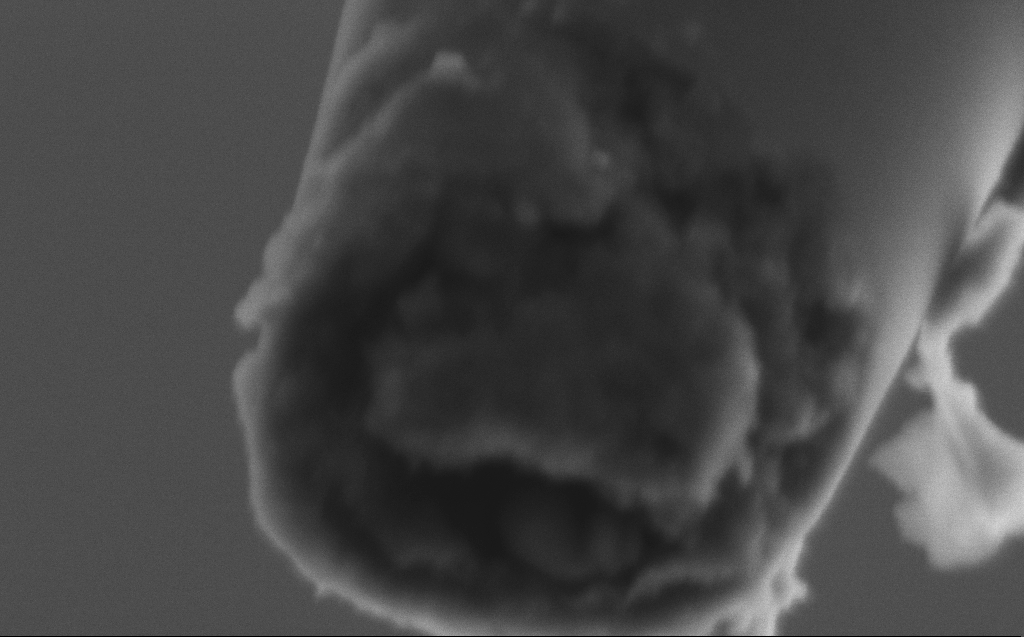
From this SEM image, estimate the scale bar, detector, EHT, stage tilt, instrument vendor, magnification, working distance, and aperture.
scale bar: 200 nm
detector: SE2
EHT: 2 kV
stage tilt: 45°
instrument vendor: Zeiss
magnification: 250 K X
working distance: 5 mm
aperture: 30 µm